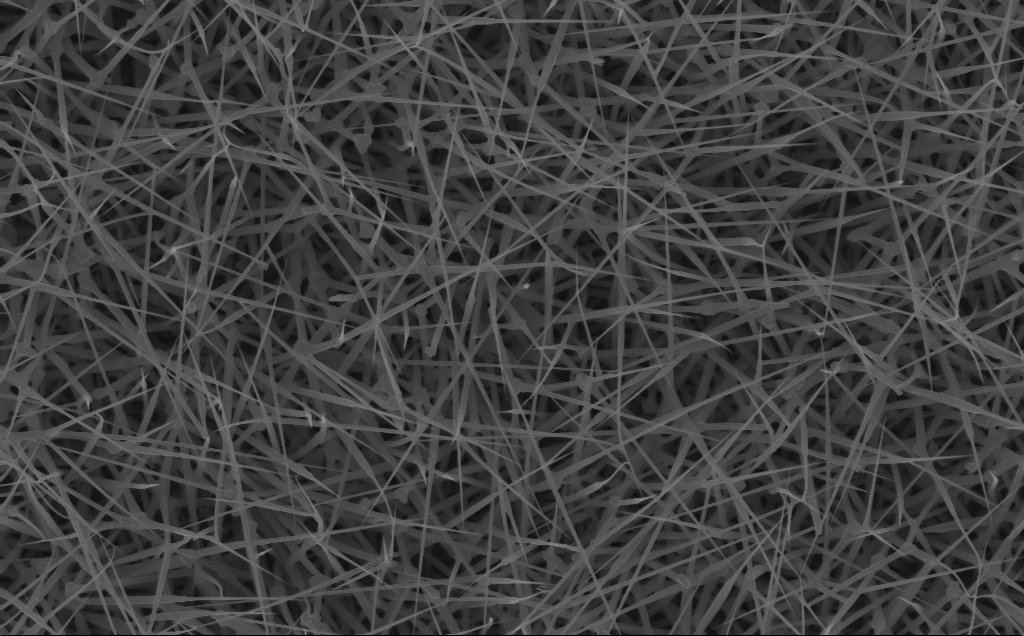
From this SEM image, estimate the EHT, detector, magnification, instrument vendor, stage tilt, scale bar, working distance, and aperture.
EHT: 10 kV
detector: InLens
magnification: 20 K X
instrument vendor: Zeiss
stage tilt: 0°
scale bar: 2000 nm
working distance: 6 mm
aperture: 30 µm